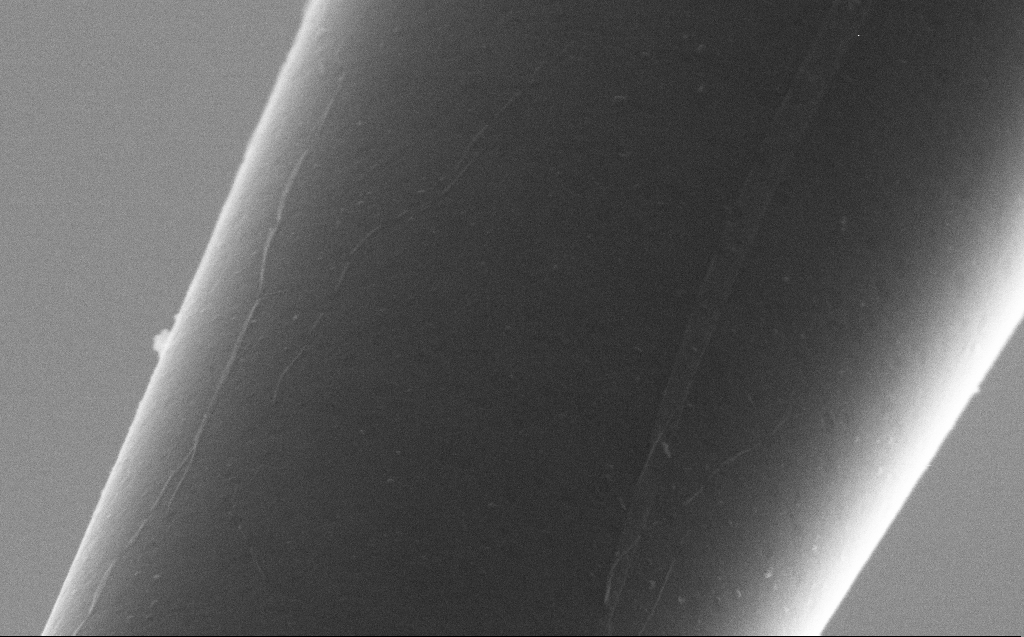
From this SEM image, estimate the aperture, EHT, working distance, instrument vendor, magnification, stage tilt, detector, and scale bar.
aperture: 30 µm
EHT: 5 kV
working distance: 6 mm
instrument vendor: Zeiss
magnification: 100 K X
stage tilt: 45°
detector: SE2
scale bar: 200 nm